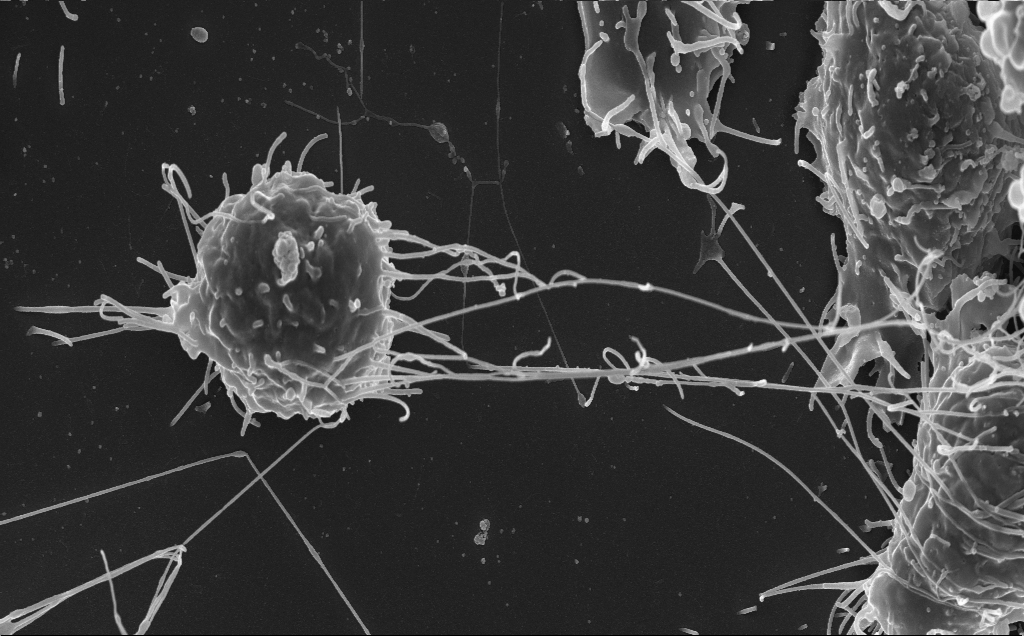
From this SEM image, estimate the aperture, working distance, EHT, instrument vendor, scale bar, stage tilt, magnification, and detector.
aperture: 30 µm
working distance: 5 mm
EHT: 10 kV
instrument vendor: Zeiss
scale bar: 2000 nm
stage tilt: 0.7°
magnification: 9.05 K X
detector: InLens